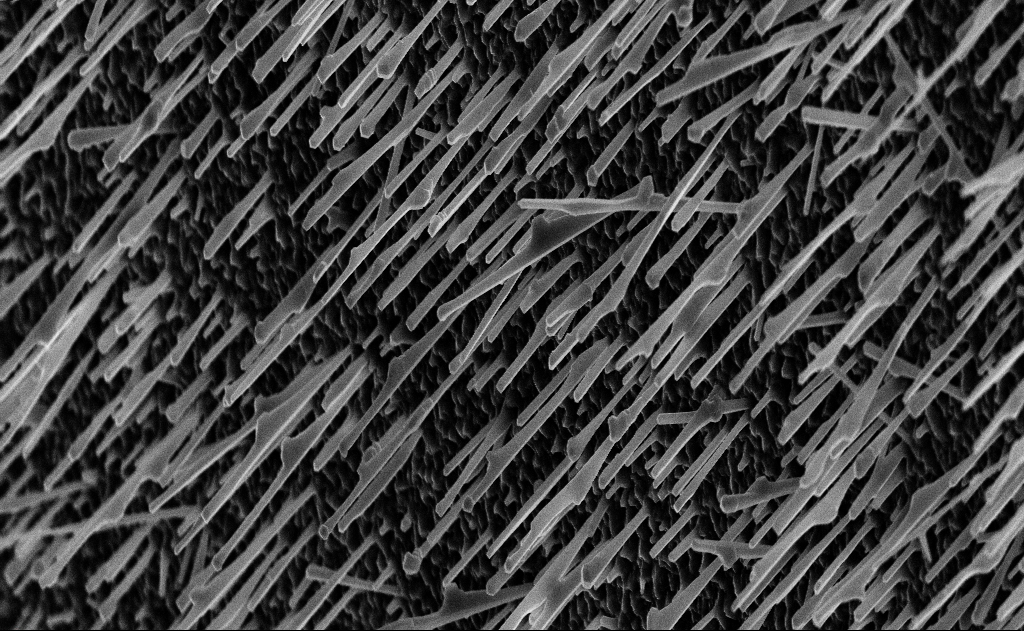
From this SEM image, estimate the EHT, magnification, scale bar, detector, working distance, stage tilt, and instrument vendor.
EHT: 10 kV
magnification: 10 K X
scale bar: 2000 nm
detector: InLens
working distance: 15 mm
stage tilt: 0°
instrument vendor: Zeiss